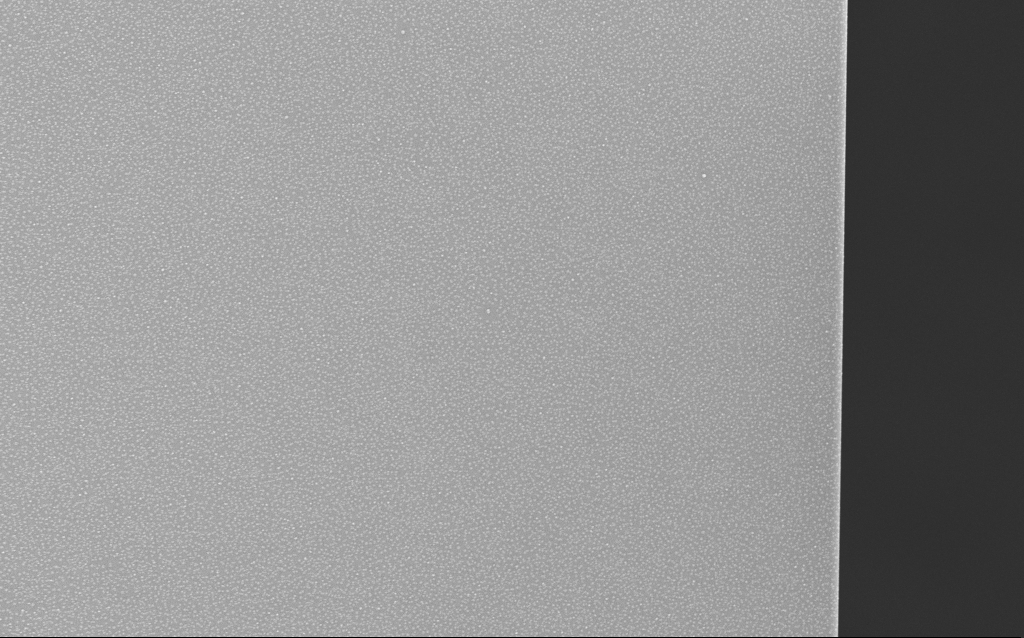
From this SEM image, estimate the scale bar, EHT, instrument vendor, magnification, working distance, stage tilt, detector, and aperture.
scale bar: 20000 nm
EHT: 20 kV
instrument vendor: Zeiss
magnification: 1 K X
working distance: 5.4 mm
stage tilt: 0°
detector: InLens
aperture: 30 µm